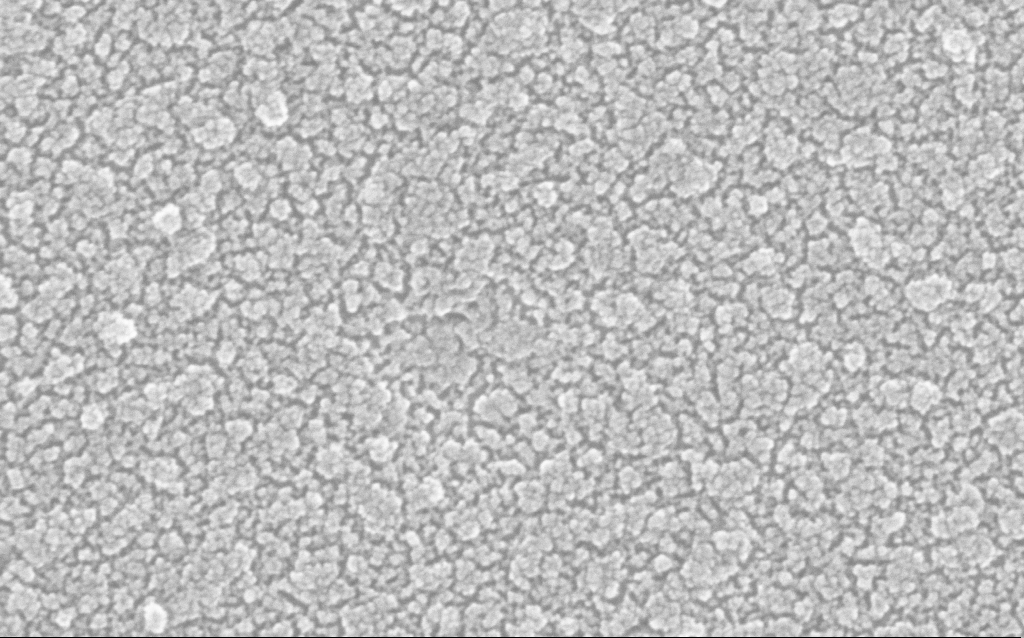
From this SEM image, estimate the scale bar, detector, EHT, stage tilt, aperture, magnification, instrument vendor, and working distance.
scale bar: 100 nm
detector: InLens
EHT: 20 kV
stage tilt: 0°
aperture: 30 µm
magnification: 500 K X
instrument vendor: Zeiss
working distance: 1.9 mm